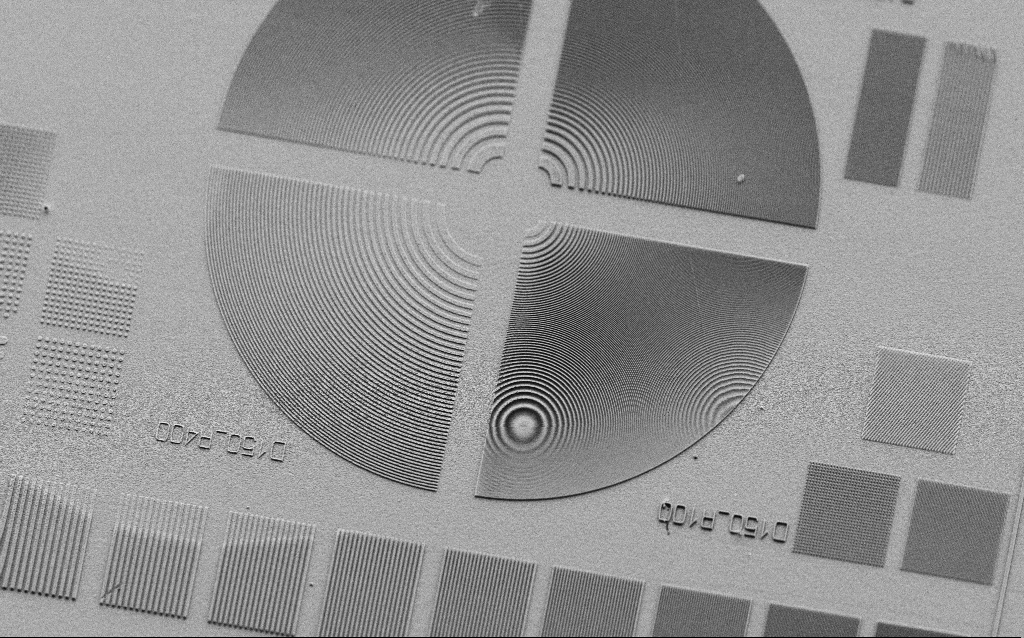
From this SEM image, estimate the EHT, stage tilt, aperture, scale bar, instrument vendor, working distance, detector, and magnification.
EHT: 3 kV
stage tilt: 45°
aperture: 30 µm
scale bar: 10000 nm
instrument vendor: Zeiss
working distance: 5 mm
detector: SE2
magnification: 1.41 K X